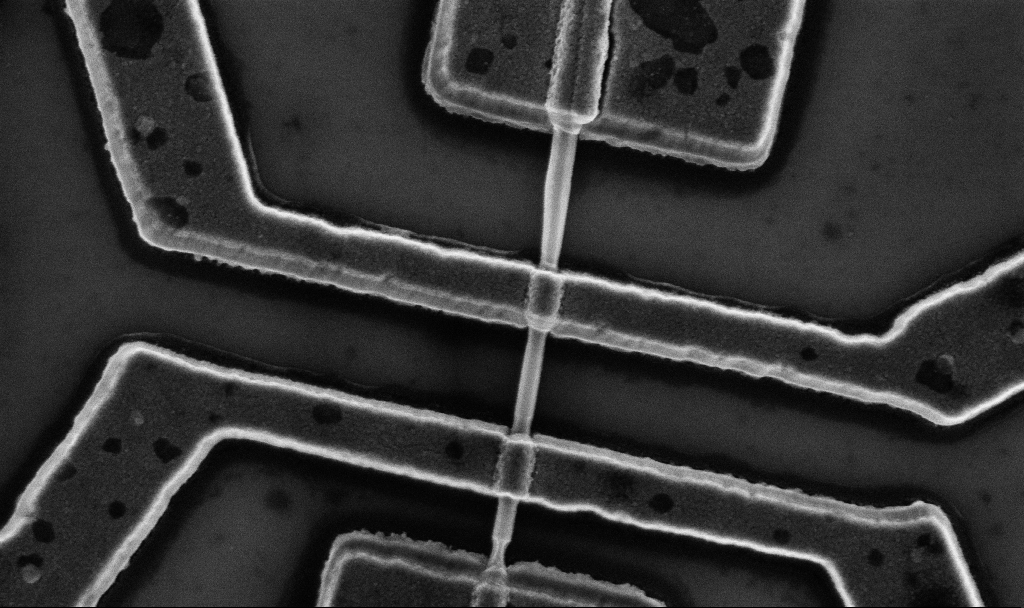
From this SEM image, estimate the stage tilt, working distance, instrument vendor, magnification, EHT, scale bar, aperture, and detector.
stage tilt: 0°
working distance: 10.7 mm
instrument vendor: Zeiss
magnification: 60 K X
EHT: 5 kV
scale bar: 1000 nm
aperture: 30 µm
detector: InLens